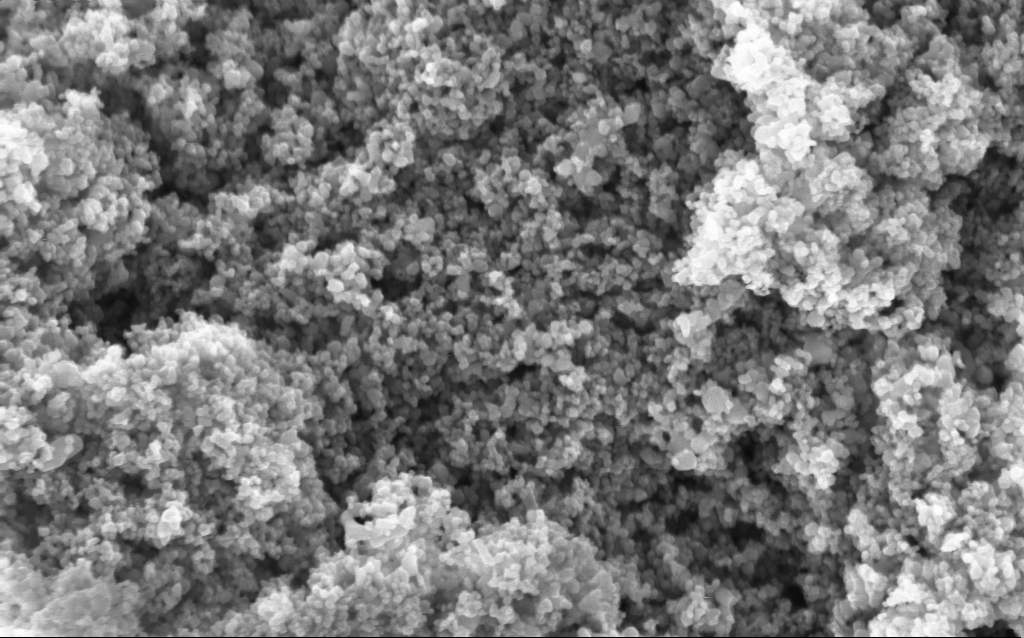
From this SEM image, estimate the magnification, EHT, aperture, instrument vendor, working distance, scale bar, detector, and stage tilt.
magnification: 114.73 K X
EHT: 5 kV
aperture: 30 µm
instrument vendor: Zeiss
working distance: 4.4 mm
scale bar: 200 nm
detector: InLens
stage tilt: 0°